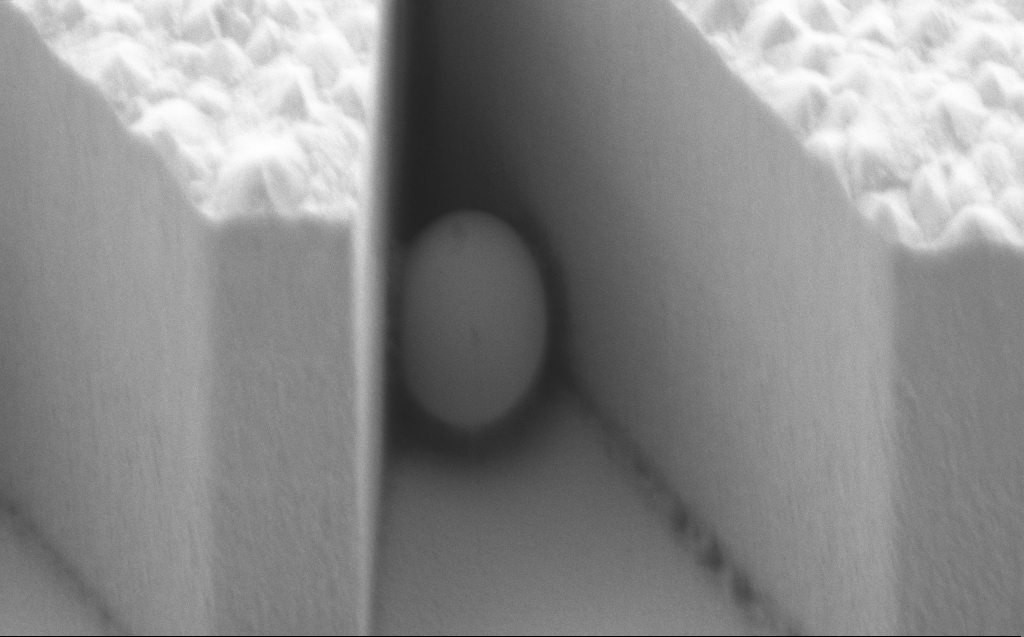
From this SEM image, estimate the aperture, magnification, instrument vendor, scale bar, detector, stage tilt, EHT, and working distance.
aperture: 120 µm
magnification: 21.84 K X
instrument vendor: Zeiss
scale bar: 2000 nm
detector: SE2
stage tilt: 45.1°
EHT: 3 kV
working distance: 7 mm